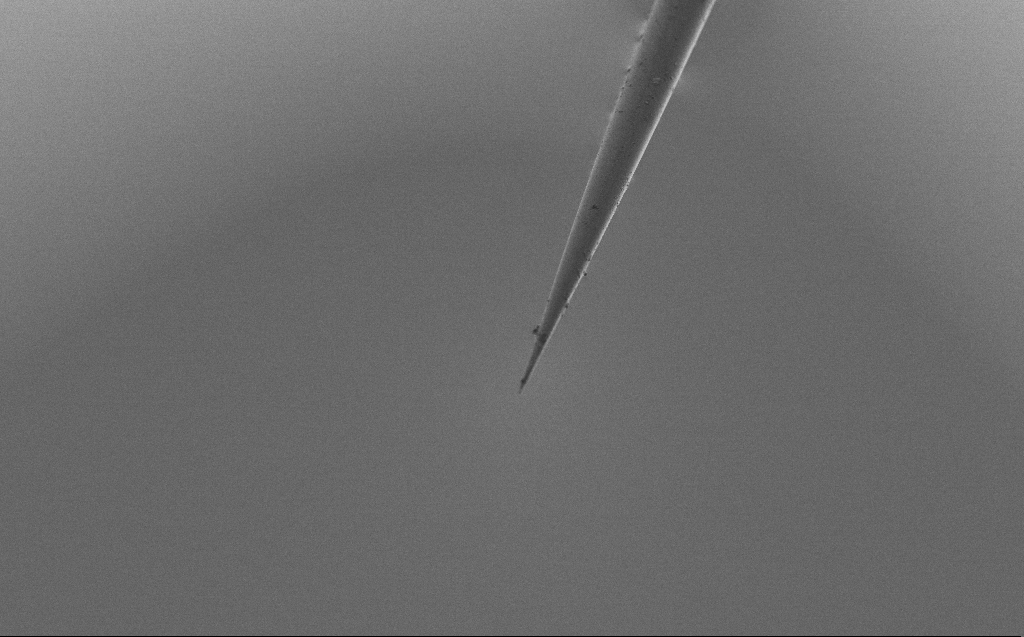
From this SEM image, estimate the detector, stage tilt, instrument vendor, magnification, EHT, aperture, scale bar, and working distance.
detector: SE2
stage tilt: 45°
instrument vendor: Zeiss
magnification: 1 K X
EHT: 2 kV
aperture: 30 µm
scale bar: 20000 nm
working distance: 3 mm